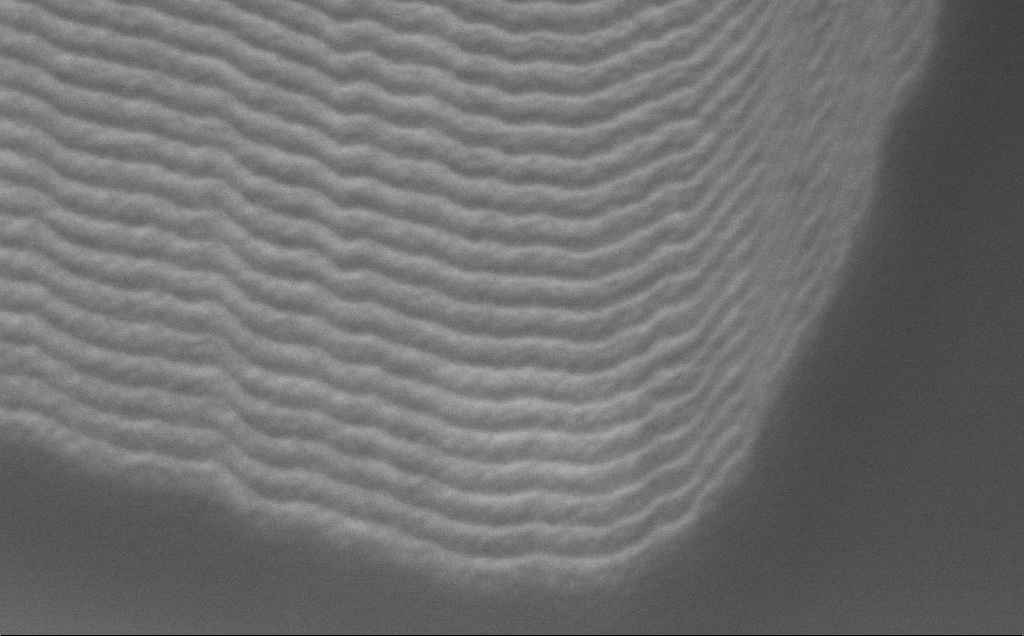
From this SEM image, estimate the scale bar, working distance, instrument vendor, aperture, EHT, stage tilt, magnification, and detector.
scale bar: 2000 nm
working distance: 8 mm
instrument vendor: Zeiss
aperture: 30 µm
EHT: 1.2 kV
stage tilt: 45°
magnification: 32.57 K X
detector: InLens